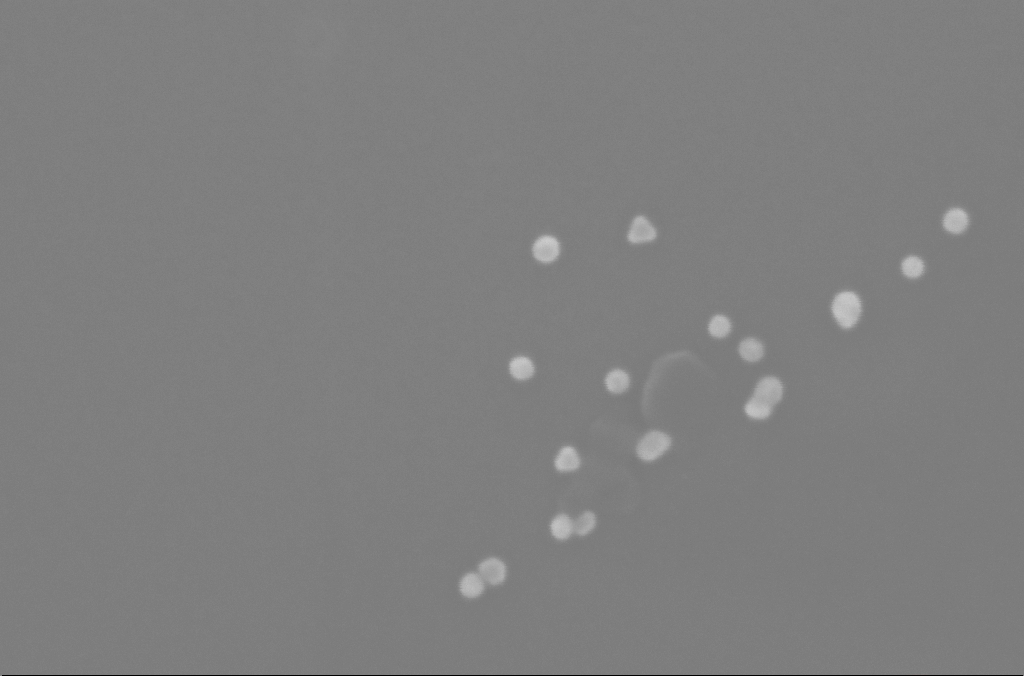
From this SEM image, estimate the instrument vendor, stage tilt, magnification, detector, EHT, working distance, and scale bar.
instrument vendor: Zeiss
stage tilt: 0°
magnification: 435.02 K X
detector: InLens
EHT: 10 kV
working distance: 2.5 mm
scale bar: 100 nm